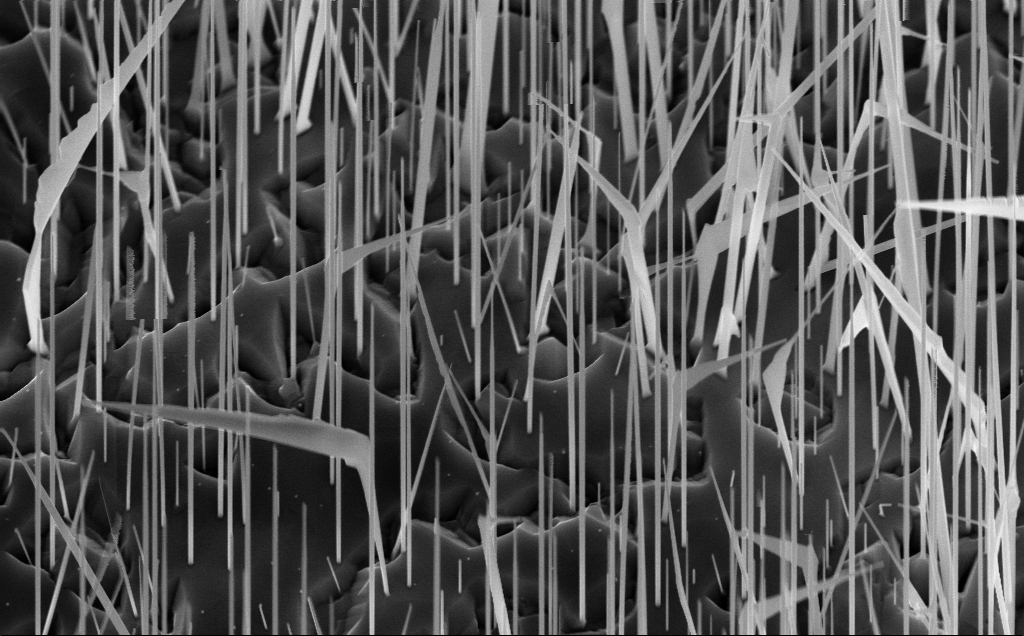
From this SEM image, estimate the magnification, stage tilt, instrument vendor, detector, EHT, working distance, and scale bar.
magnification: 22.49 K X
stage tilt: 45°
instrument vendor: Zeiss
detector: InLens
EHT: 10 kV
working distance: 7 mm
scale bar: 2000 nm